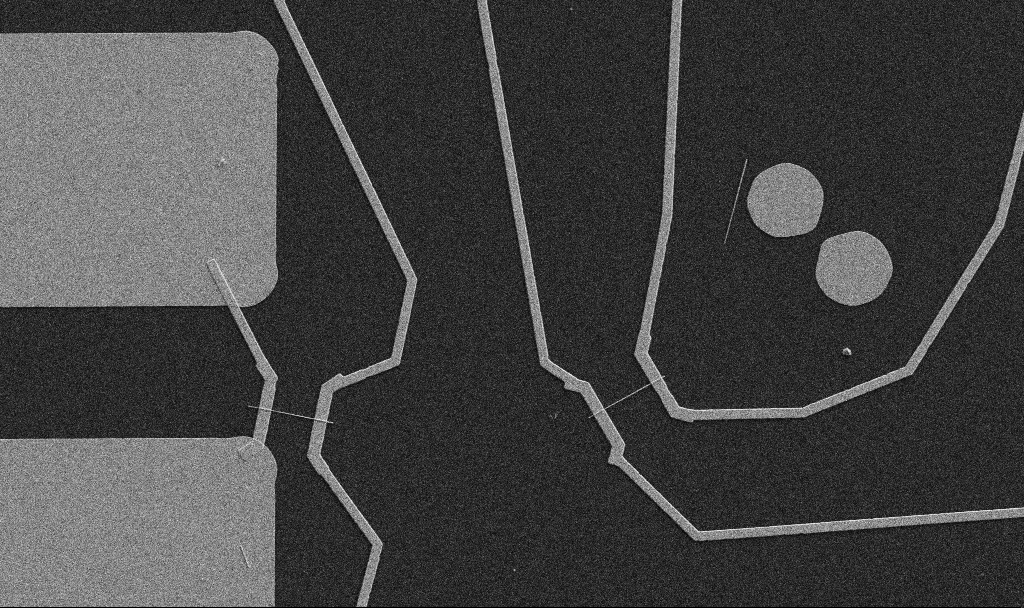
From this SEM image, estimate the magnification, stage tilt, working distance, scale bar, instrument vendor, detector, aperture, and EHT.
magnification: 5 K X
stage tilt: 0°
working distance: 10.7 mm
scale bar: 10000 nm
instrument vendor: Zeiss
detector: SE2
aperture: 30 µm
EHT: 5 kV